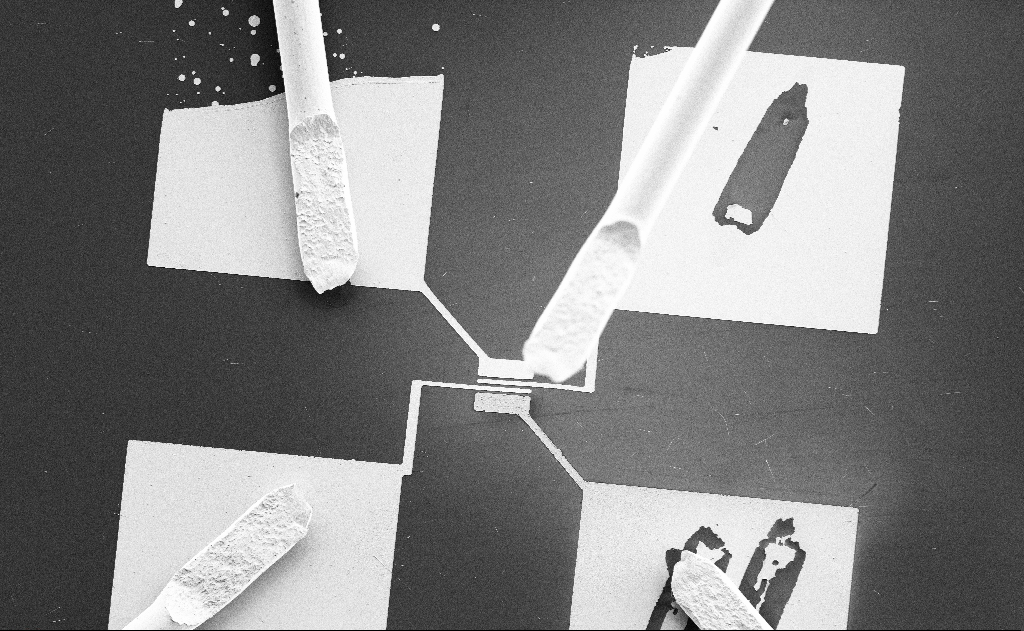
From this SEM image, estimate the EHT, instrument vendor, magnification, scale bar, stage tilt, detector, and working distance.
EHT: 5 kV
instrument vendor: Zeiss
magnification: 0.684 K X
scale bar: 100000 nm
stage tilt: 0°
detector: SE2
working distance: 15 mm